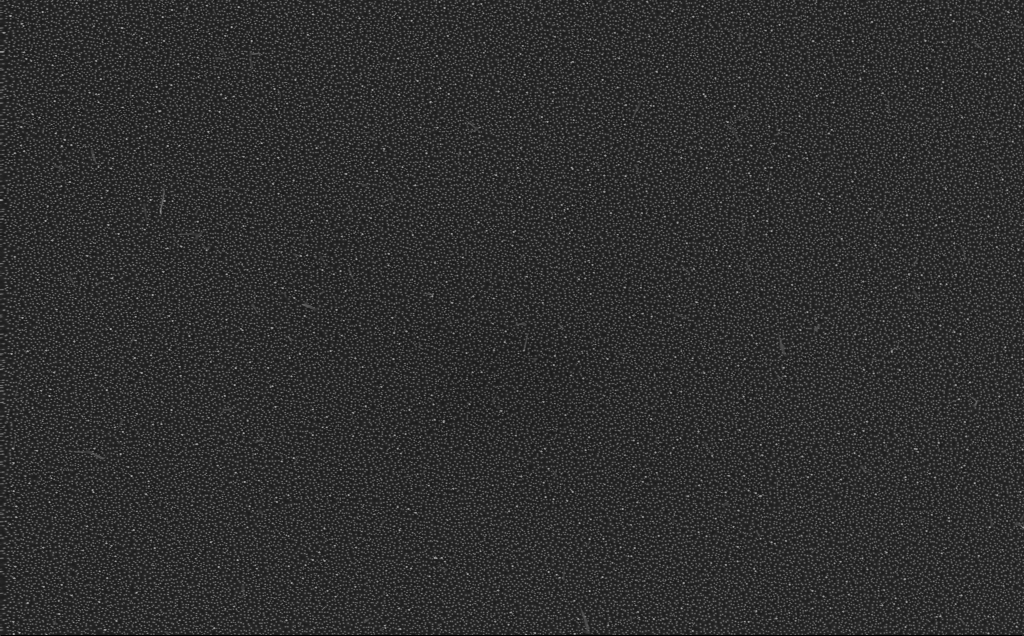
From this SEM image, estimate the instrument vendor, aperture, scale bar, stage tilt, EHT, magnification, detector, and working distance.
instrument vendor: Zeiss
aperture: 30 µm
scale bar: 2000 nm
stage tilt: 0°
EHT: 10 kV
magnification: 10 K X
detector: InLens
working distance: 4 mm